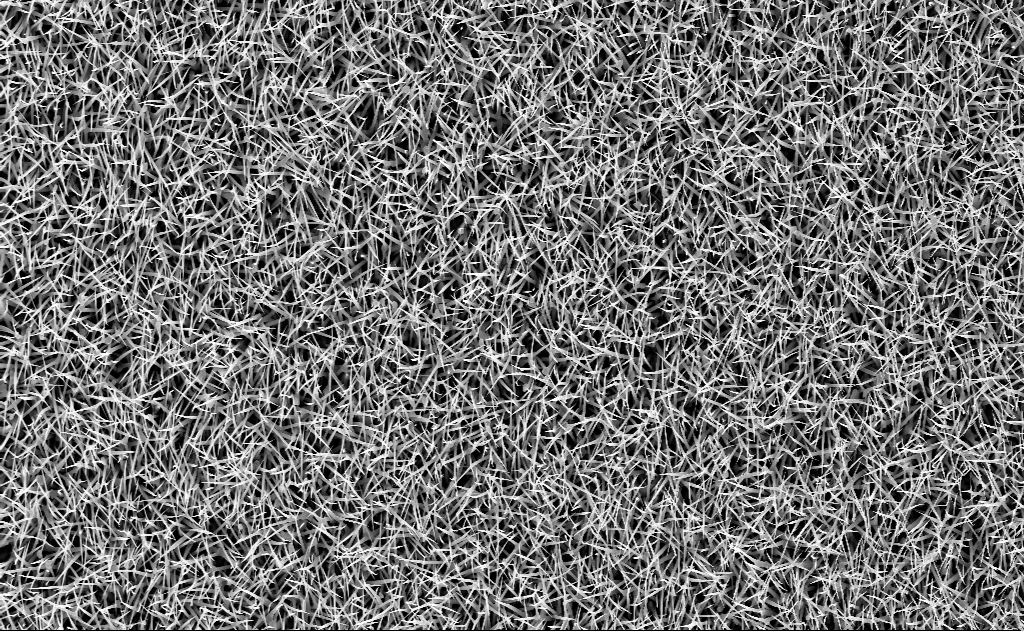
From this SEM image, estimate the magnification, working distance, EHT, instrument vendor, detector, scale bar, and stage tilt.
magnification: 5 K X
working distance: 7 mm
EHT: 10 kV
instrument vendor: Zeiss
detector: InLens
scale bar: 10000 nm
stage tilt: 0°